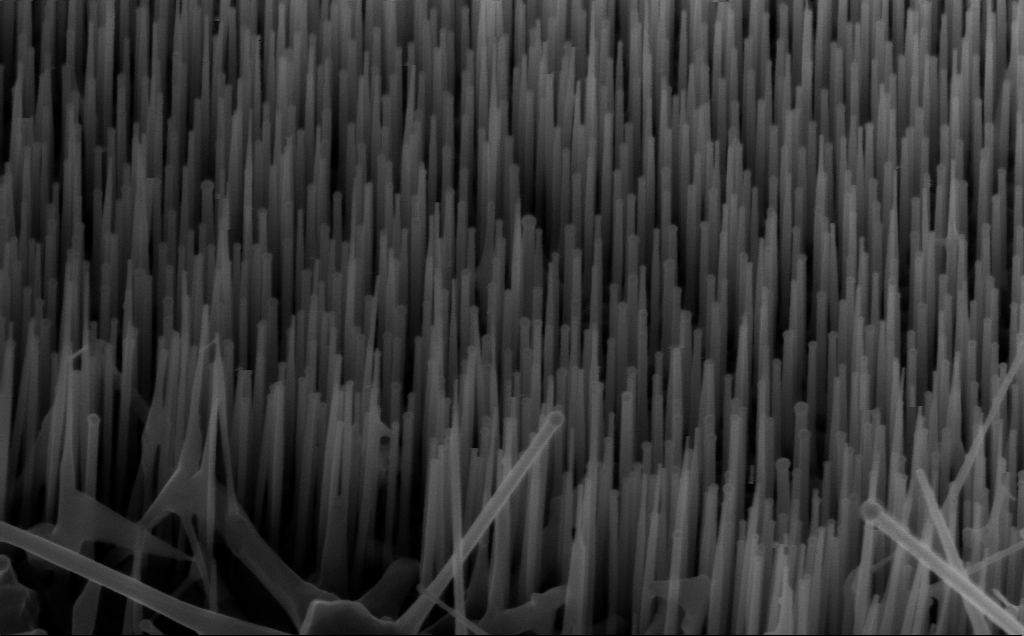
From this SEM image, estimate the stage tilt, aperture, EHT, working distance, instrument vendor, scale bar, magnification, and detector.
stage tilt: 45°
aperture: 30 µm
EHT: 10 kV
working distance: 5 mm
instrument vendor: Zeiss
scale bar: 200 nm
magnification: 80 K X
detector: InLens